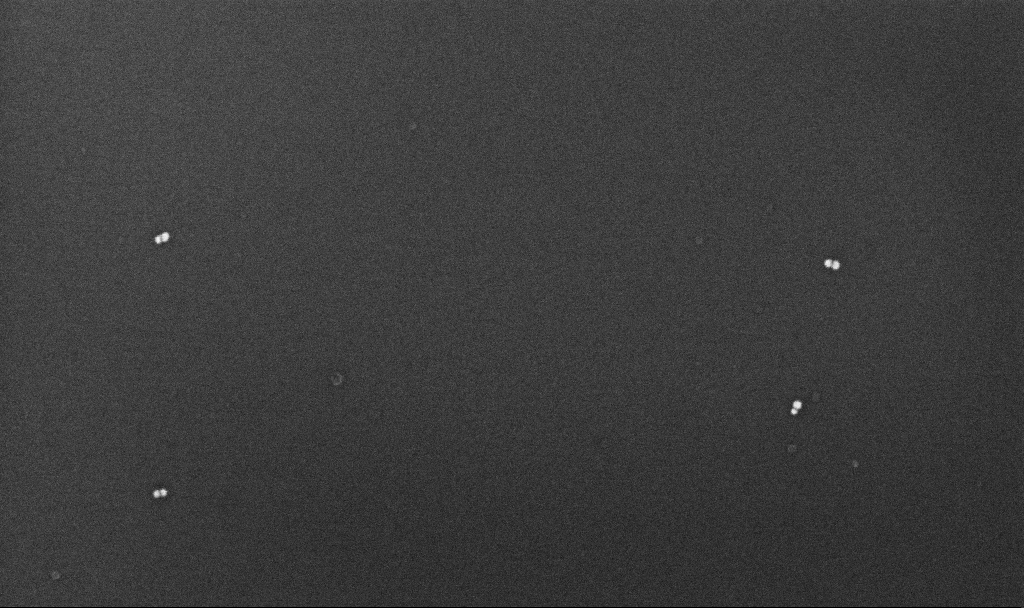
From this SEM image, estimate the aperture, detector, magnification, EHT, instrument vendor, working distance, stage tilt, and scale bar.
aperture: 30 µm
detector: InLens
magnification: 175.93 K X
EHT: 10 kV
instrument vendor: Zeiss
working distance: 4.3 mm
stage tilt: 0°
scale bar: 200 nm